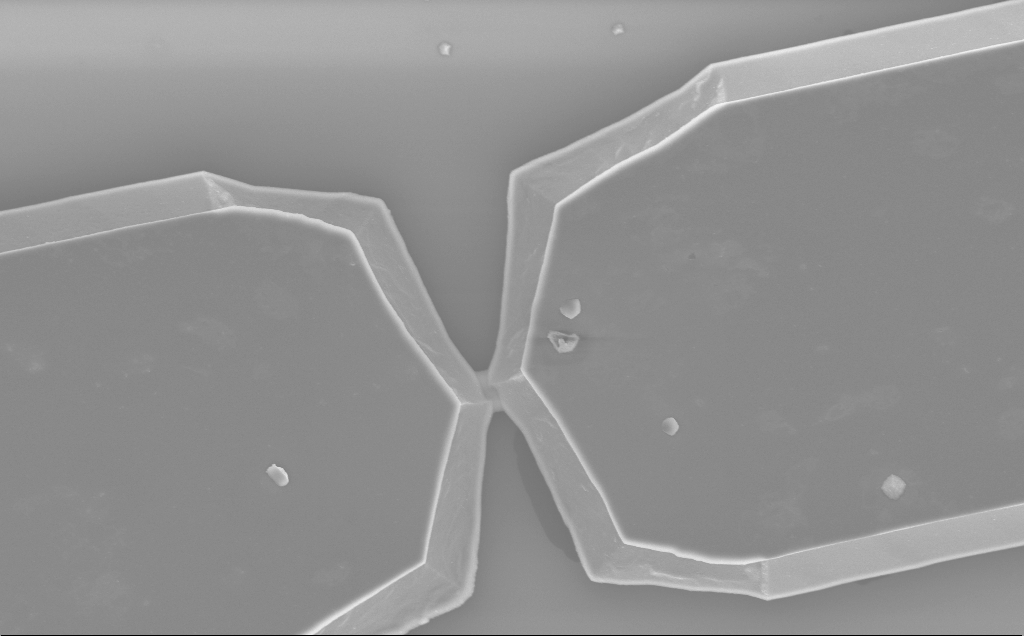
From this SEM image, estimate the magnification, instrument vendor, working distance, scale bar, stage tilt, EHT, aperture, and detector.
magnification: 8.75 K X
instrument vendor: Zeiss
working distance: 10 mm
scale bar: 2000 nm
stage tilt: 0°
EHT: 5 kV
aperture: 30 µm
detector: InLens